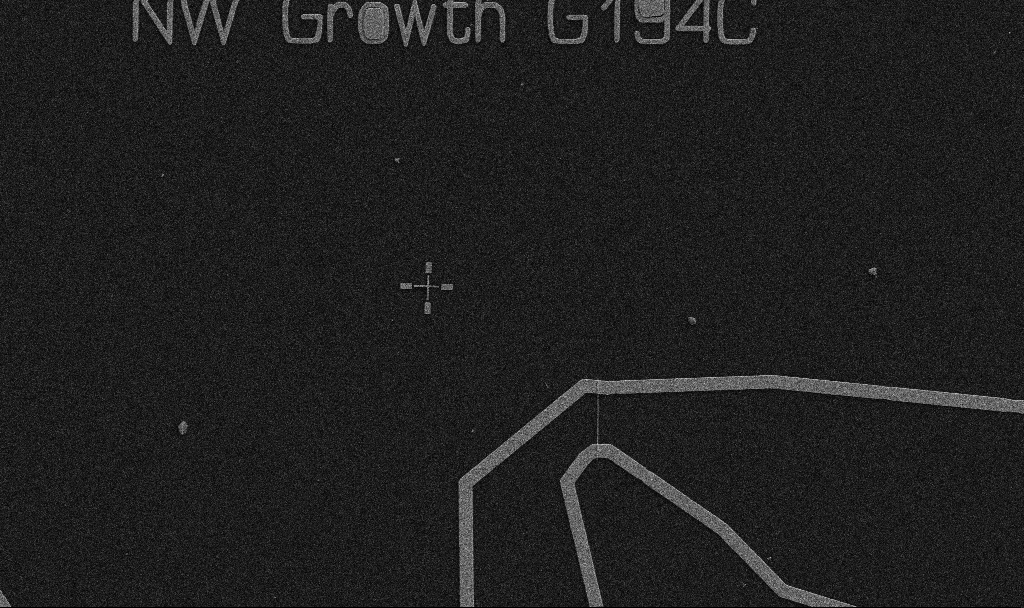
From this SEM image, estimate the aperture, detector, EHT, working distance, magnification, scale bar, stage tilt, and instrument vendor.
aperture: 30 µm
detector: SE2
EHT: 5 kV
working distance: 10.7 mm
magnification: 5 K X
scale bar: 10000 nm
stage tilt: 0°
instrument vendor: Zeiss